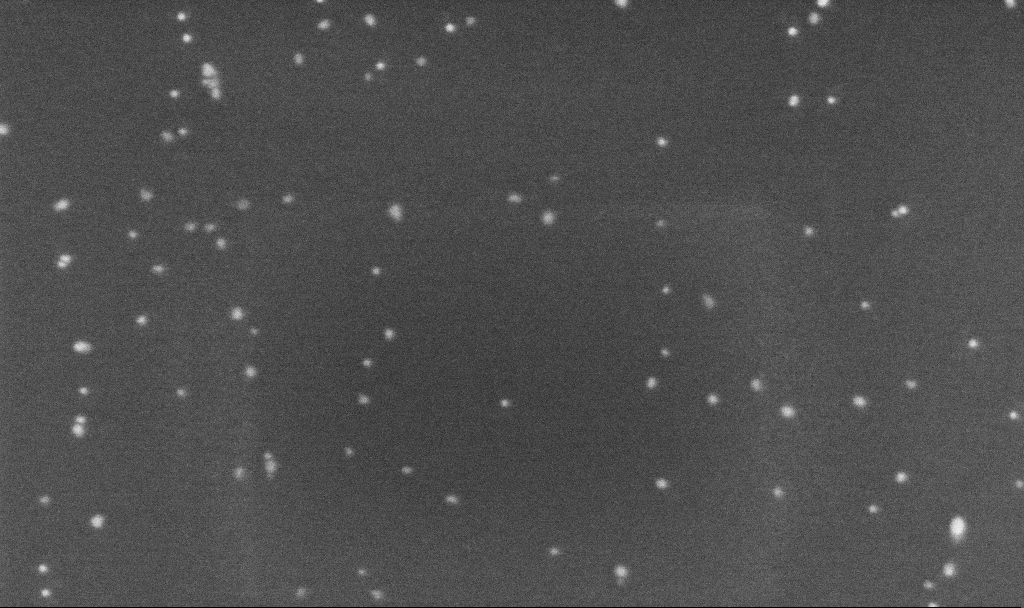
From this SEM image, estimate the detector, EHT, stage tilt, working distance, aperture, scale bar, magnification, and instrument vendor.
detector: InLens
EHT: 5 kV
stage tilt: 0°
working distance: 3.2 mm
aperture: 30 µm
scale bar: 100 nm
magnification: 250 K X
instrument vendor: Zeiss